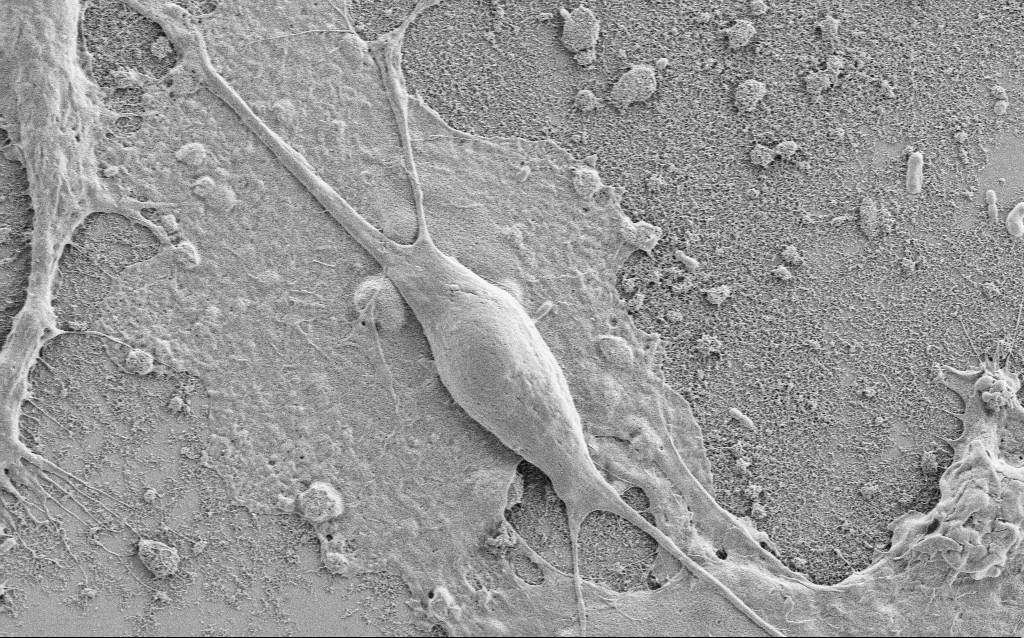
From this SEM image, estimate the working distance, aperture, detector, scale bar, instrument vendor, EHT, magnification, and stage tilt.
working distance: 6.8 mm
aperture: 30 µm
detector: SE2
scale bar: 10000 nm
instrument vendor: Zeiss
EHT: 1.5 kV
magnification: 5 K X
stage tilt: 0°